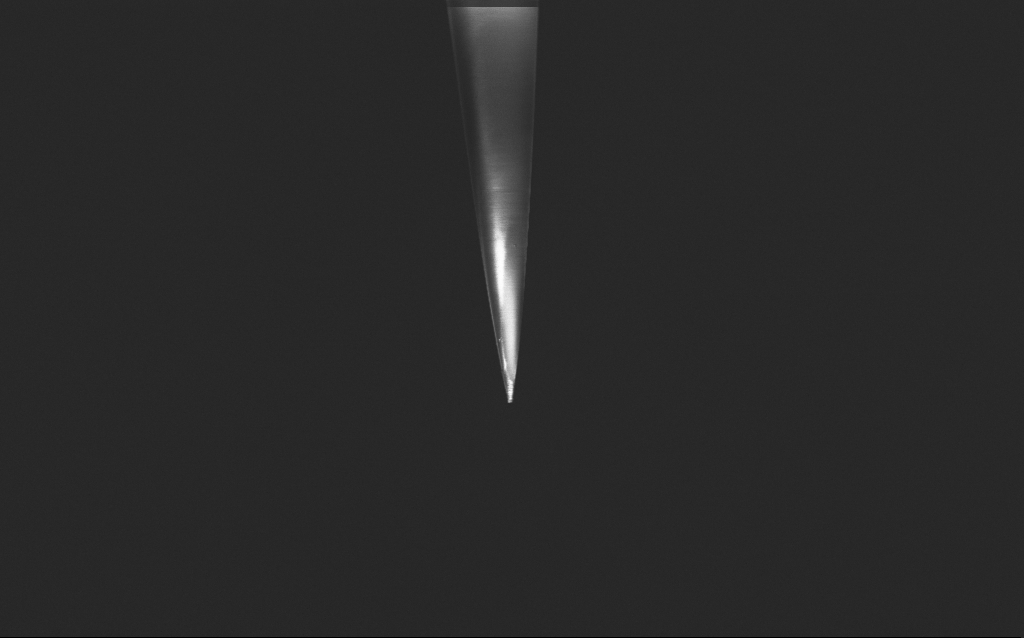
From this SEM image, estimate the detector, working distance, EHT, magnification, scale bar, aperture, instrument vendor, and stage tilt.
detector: InLens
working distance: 5 mm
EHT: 2.5 kV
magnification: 10 K X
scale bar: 2000 nm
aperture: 30 µm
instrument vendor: Zeiss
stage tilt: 45°